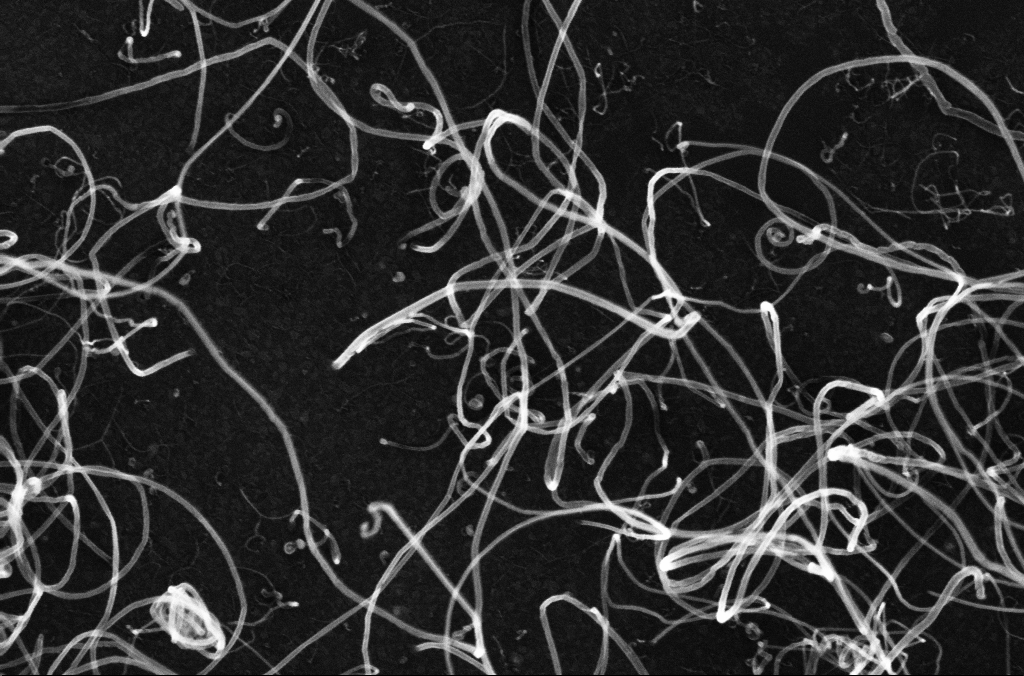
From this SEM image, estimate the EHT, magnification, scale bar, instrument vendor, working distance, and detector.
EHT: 10 kV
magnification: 100 K X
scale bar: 200 nm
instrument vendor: Zeiss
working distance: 3.3 mm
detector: InLens